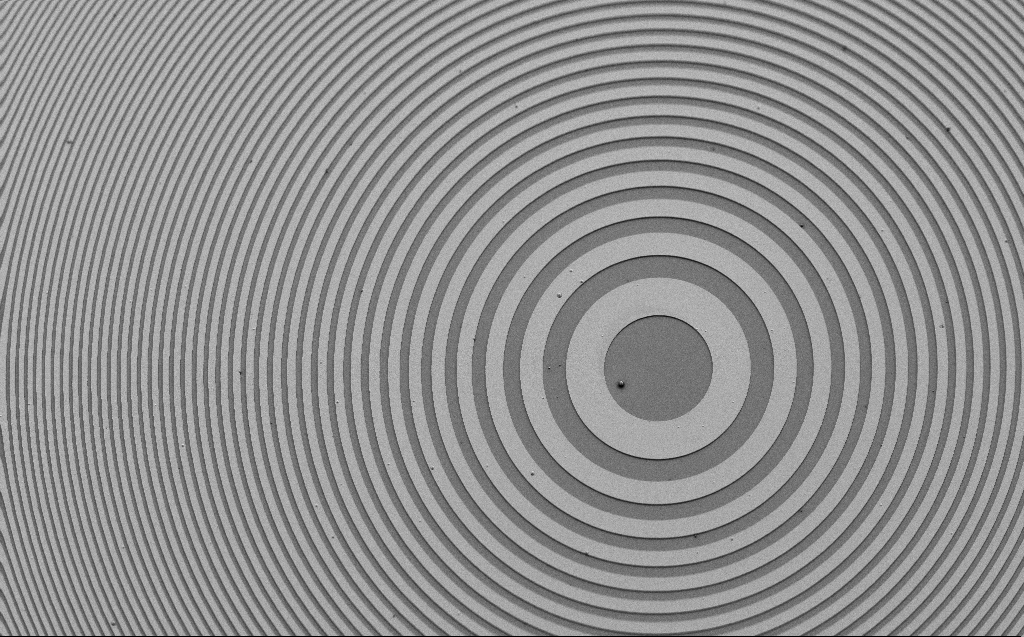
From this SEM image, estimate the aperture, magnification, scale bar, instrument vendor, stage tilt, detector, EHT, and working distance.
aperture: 30 µm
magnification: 0.603 K X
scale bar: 100000 nm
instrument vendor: Zeiss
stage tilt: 45°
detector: SE2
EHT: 5 kV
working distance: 9 mm